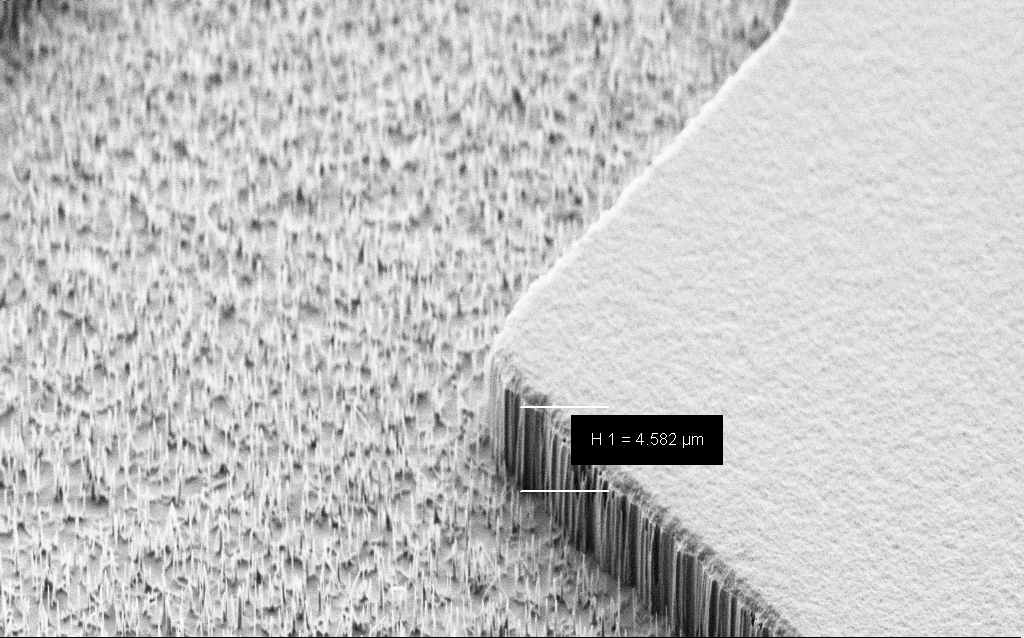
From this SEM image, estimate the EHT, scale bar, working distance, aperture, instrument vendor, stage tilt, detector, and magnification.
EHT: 2 kV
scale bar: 10000 nm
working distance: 8 mm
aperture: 30 µm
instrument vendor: Zeiss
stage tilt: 45°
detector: SE2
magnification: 6.73 K X